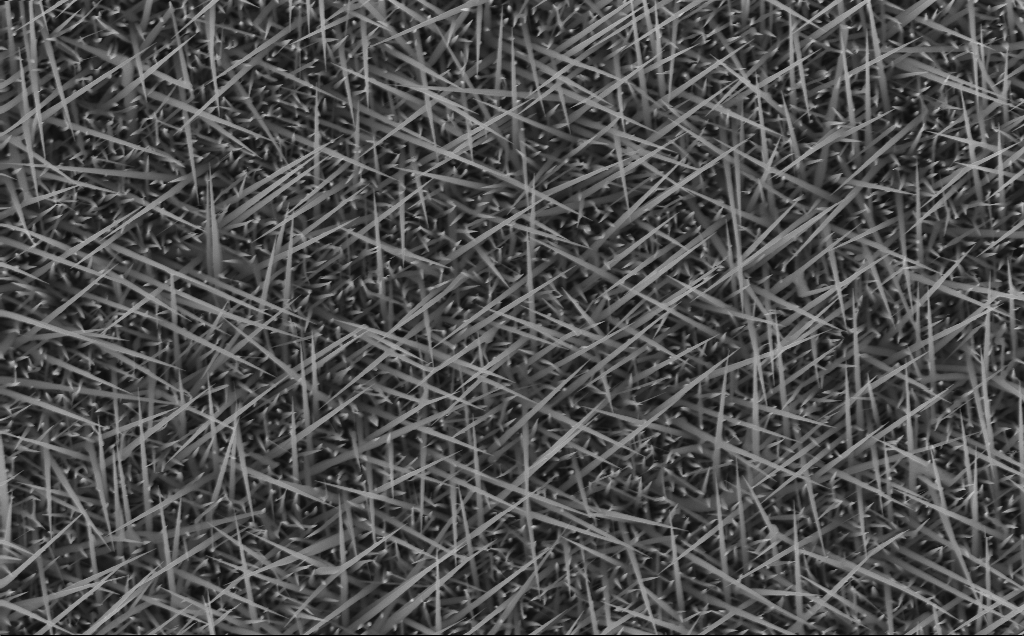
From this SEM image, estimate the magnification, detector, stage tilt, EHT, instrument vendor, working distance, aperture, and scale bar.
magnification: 40 K X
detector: InLens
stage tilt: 0°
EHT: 10 kV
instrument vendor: Zeiss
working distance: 6 mm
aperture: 30 µm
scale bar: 1000 nm